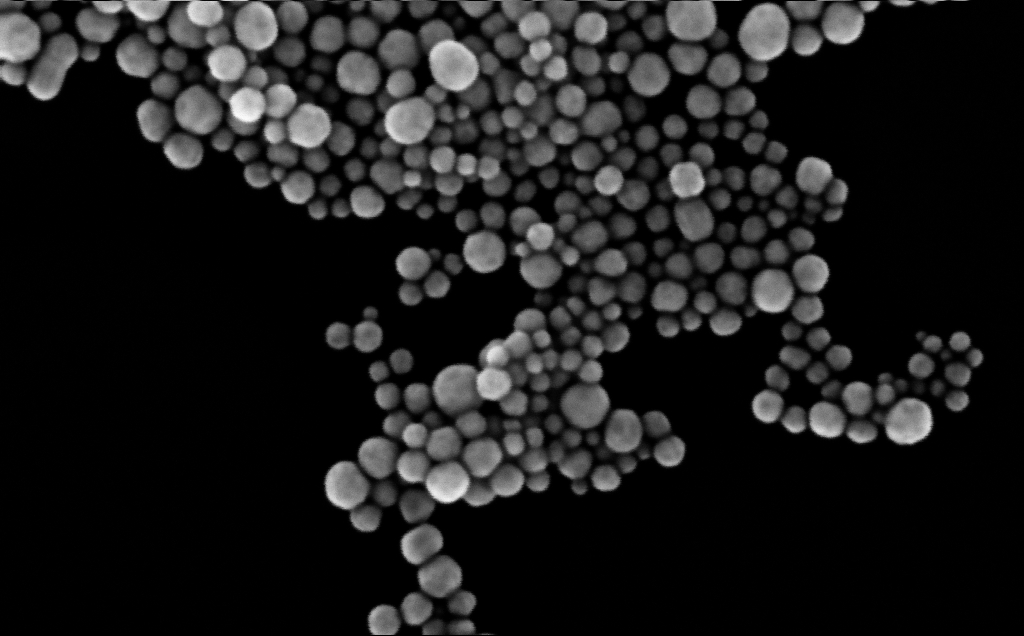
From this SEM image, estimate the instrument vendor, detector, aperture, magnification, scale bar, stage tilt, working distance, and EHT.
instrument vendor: Zeiss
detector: InLens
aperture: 30 µm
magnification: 441.57 K X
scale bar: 100 nm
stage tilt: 0°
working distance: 3 mm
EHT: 10 kV